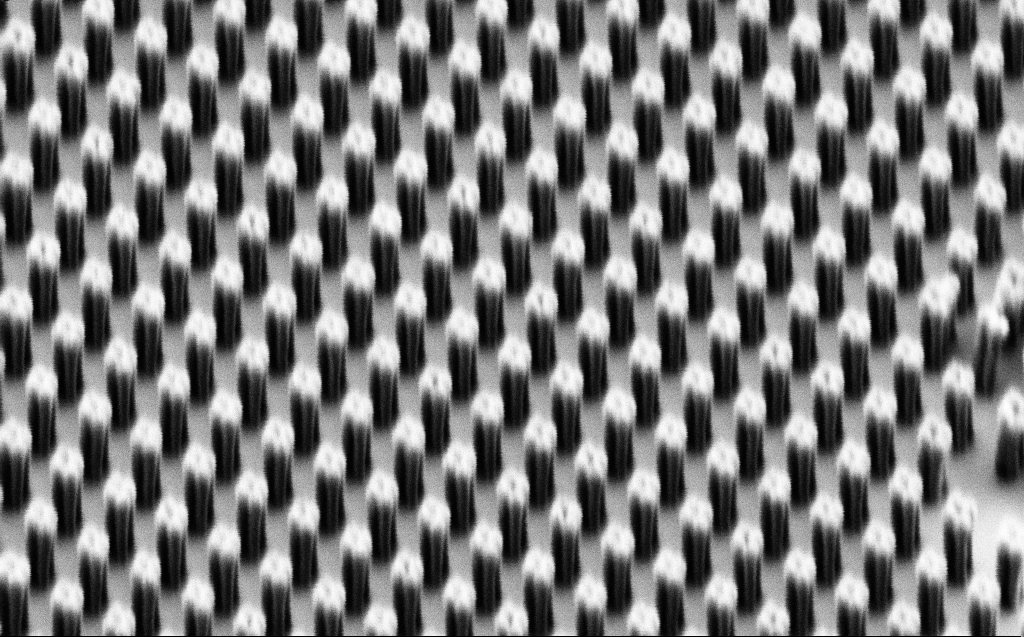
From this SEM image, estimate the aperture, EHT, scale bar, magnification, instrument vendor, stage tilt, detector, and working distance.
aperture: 30 µm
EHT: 1 kV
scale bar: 1000 nm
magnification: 43.52 K X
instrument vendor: Zeiss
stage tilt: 42.8°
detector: SE2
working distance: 6 mm